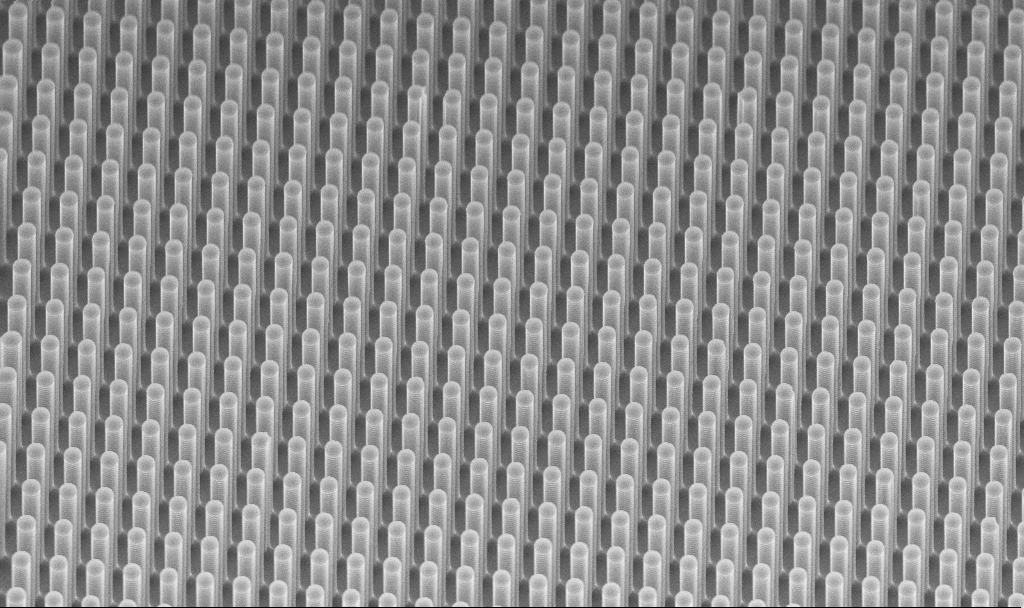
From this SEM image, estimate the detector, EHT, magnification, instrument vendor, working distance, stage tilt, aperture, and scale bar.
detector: InLens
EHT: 10 kV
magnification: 3.42 K X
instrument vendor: Zeiss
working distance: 8.2 mm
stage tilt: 45°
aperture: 30 µm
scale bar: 10000 nm